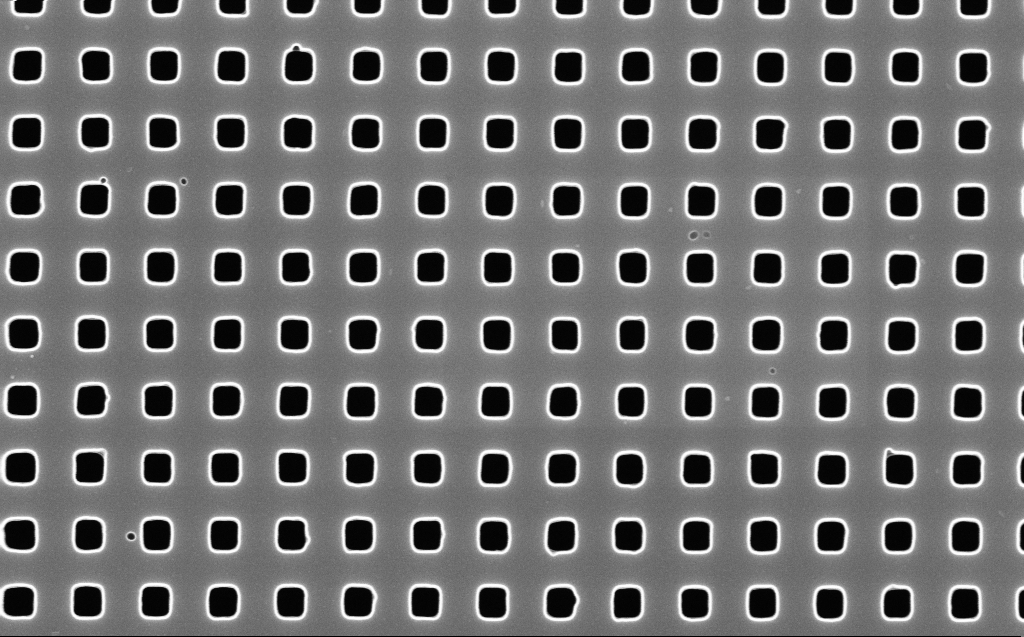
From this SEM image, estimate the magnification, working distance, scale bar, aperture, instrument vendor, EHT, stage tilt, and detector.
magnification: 50 K X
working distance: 6 mm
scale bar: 1000 nm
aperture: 30 µm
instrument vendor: Zeiss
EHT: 10 kV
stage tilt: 0°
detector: InLens